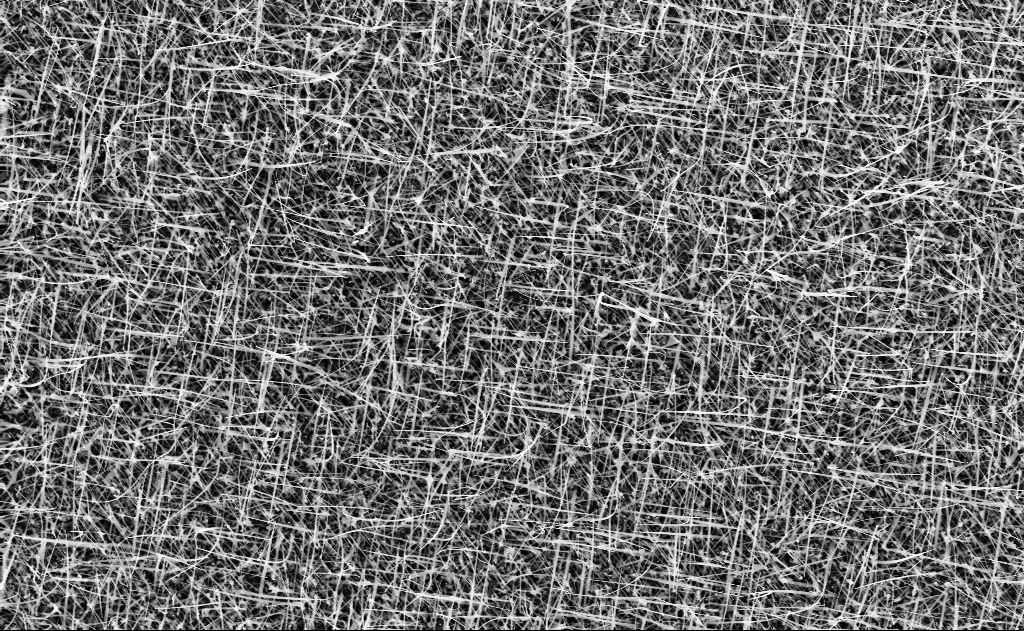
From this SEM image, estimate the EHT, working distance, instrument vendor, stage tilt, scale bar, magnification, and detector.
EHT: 10 kV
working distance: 9 mm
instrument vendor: Zeiss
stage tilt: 0°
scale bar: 2000 nm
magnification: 10 K X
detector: InLens